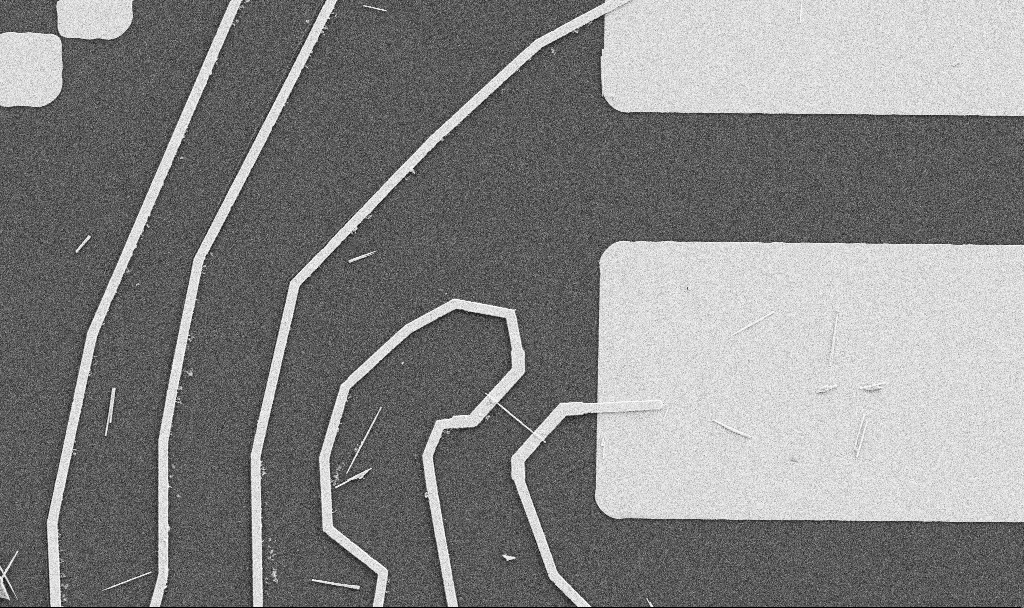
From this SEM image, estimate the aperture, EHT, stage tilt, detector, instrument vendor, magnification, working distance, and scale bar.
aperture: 30 µm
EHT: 5 kV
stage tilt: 0°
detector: SE2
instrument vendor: Zeiss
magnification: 5 K X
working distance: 10.7 mm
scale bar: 10000 nm